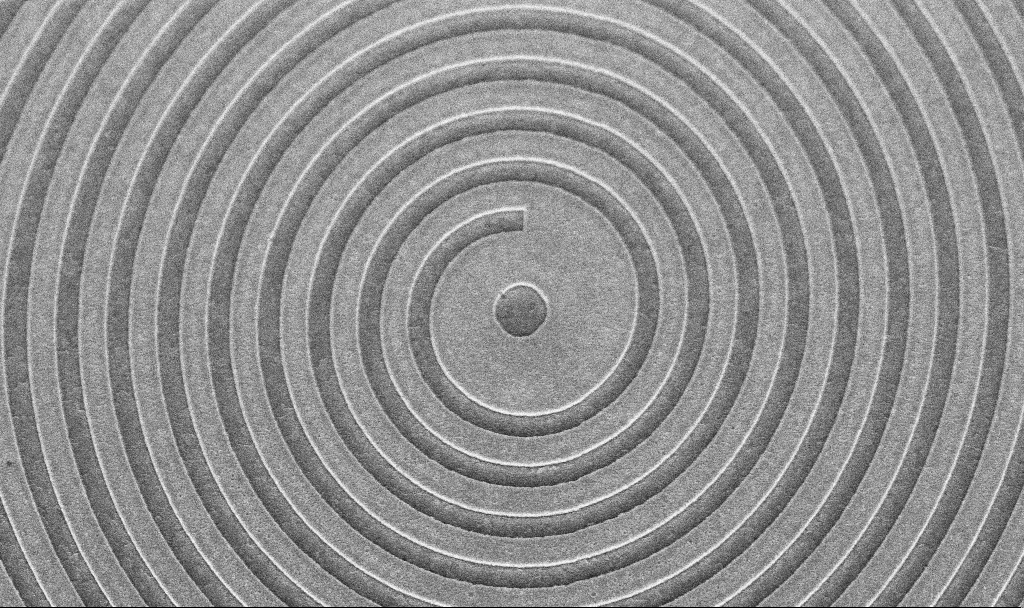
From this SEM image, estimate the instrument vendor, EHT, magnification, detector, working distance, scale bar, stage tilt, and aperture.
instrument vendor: Zeiss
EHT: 5 kV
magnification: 24.27 K X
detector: InLens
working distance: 6.3 mm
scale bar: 2000 nm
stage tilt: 45°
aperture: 30 µm